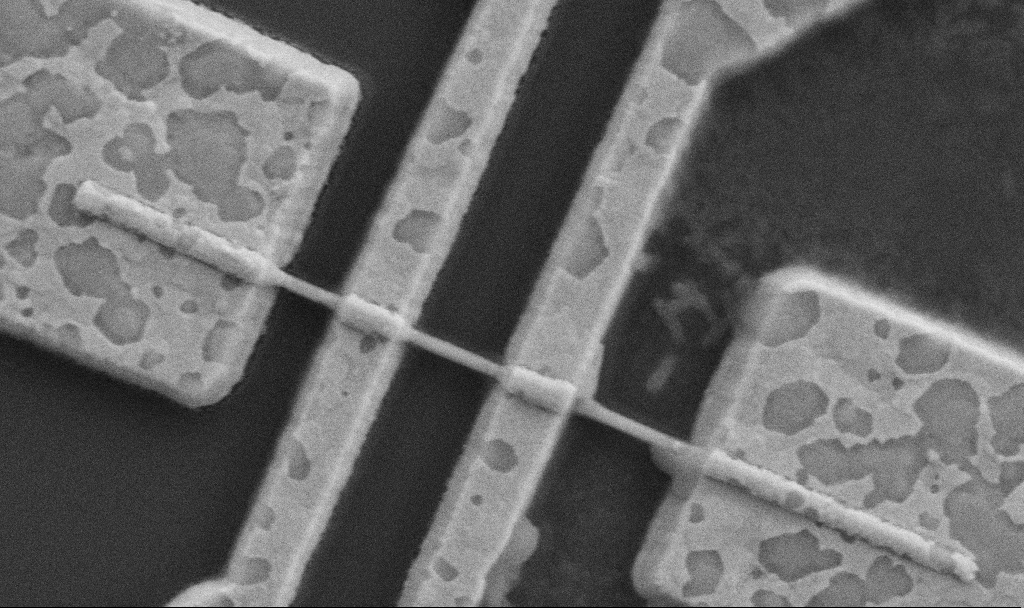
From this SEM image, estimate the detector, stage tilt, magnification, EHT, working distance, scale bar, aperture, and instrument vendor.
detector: SE2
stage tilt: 0°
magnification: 60 K X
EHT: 5 kV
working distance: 8.7 mm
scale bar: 1000 nm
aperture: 30 µm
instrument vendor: Zeiss